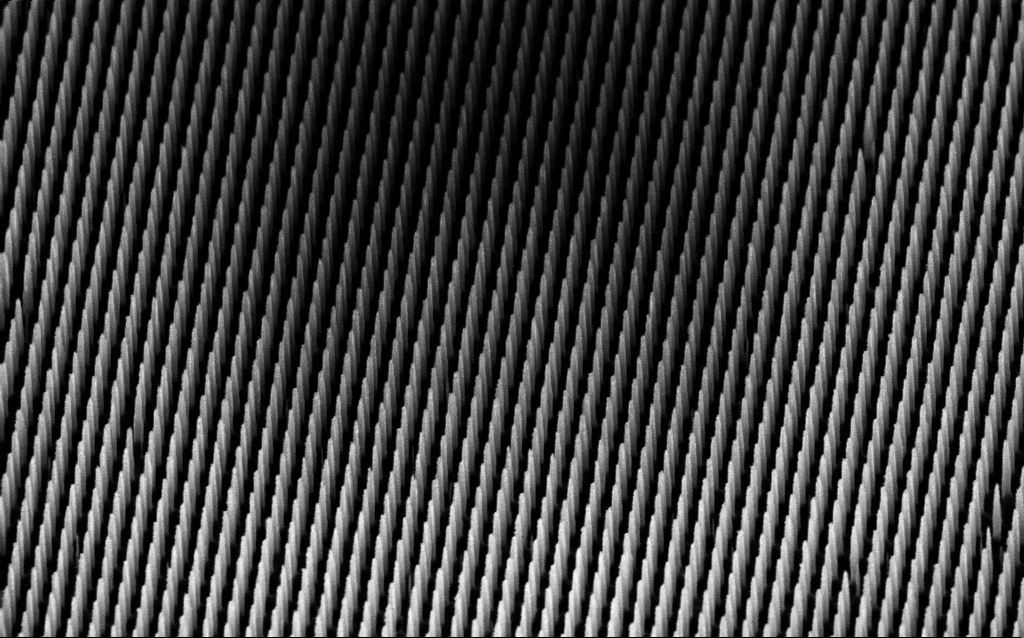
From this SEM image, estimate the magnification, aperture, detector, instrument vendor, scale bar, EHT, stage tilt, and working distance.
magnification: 41.31 K X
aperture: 30 µm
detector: InLens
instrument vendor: Zeiss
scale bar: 1000 nm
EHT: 3 kV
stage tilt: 45°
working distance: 5 mm